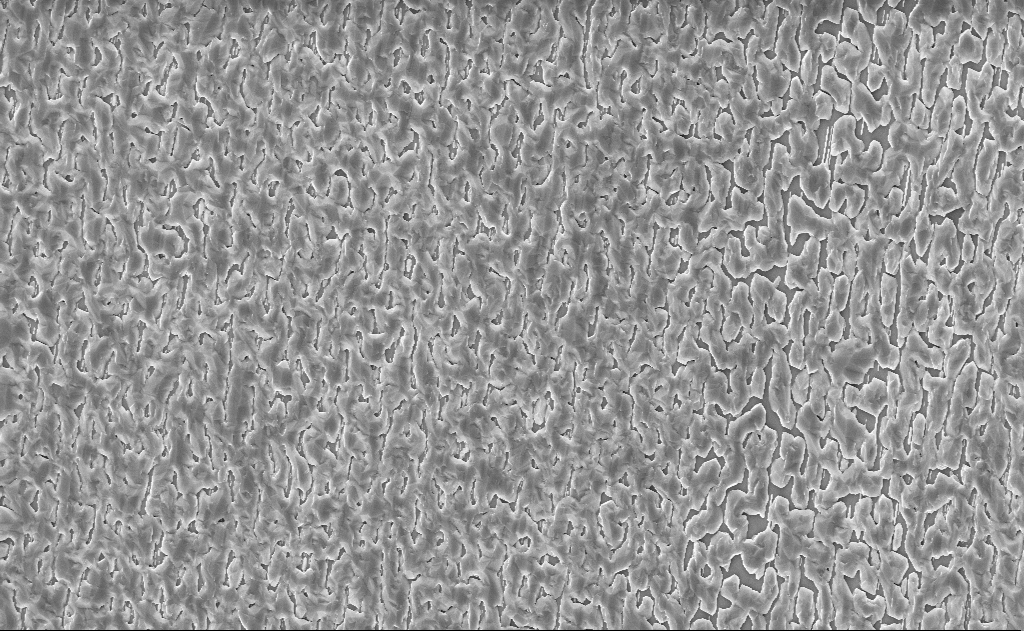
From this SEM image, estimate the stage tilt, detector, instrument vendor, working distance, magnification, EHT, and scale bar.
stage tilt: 0°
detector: InLens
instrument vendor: Zeiss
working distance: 14 mm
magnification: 5 K X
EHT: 10 kV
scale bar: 10000 nm